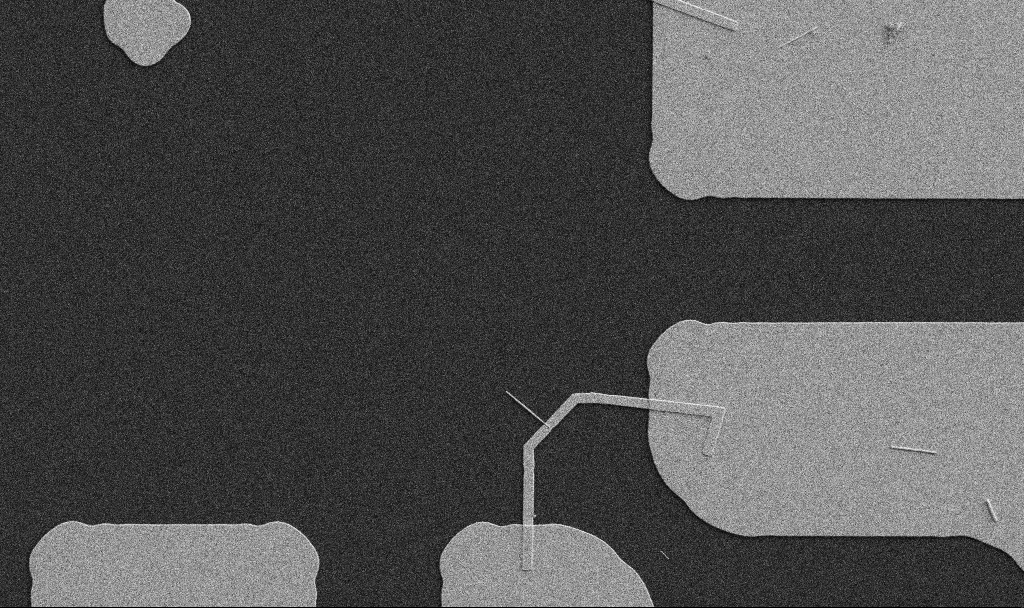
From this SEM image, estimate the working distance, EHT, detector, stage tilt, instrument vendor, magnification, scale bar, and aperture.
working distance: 10.7 mm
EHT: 5 kV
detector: SE2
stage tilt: -0°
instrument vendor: Zeiss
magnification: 5 K X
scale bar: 10000 nm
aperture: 30 µm